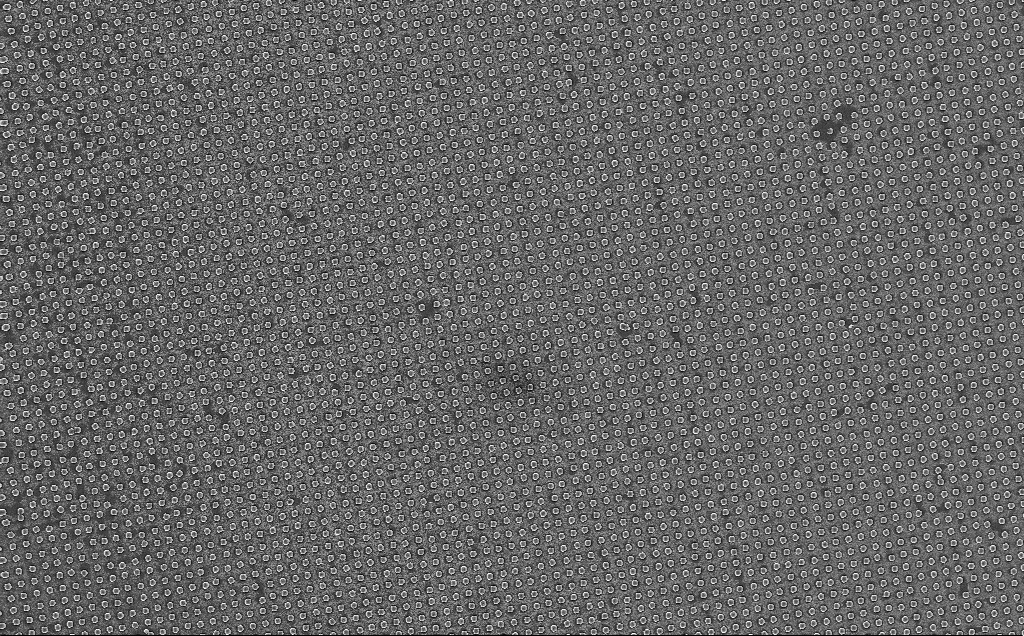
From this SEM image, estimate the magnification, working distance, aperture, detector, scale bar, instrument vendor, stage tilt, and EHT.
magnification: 3.14 K X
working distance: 7 mm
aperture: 30 µm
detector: InLens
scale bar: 10000 nm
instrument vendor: Zeiss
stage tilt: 0°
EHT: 5 kV